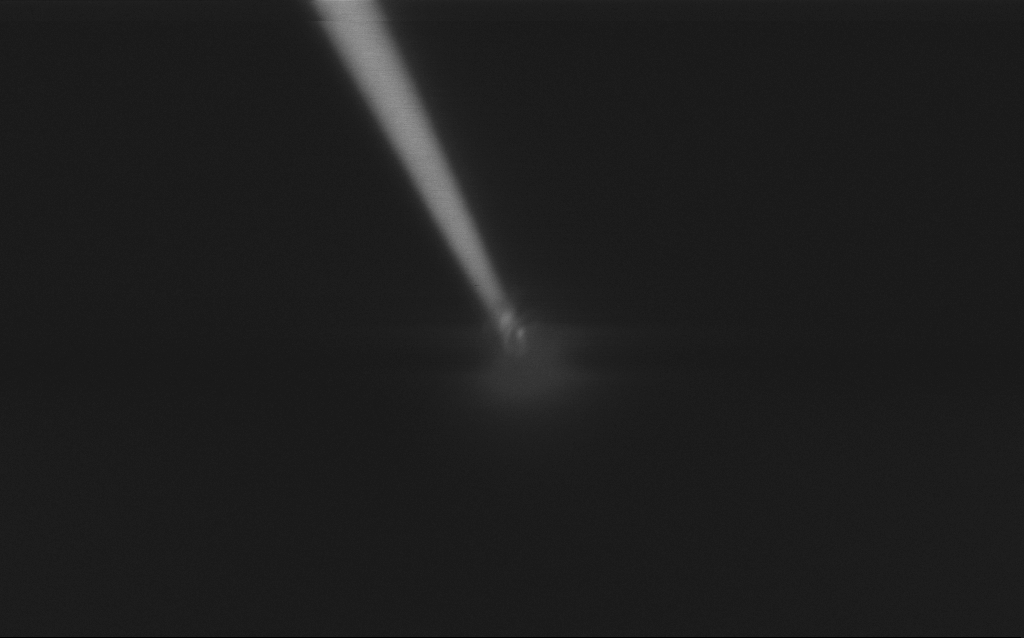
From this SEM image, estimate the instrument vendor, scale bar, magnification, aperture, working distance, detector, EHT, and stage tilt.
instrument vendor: Zeiss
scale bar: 200 nm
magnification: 100 K X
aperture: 30 µm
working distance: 7 mm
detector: InLens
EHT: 1 kV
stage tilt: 45°